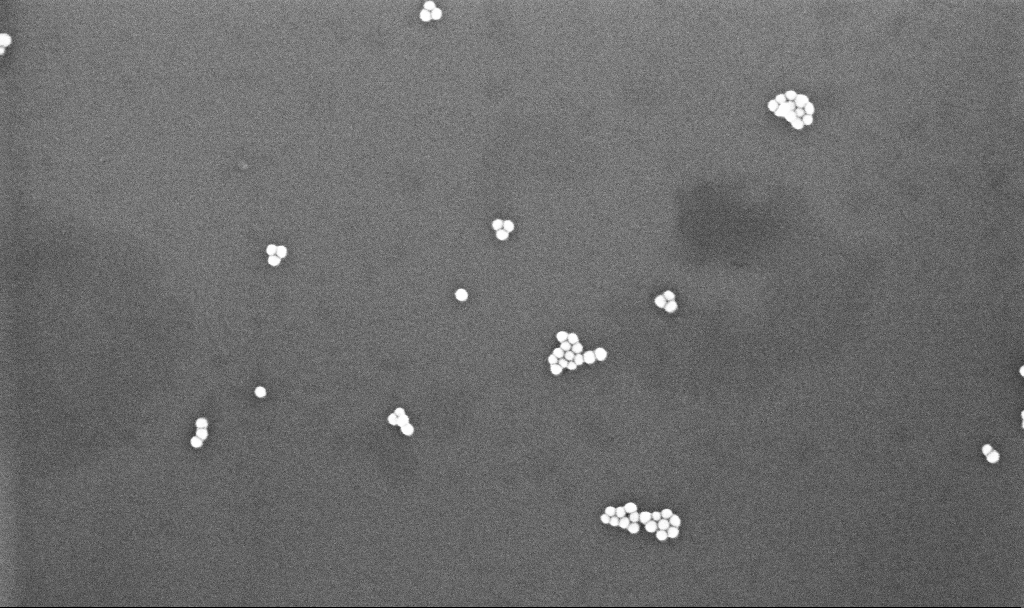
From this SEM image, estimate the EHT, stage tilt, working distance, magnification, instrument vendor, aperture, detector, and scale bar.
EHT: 10 kV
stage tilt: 0°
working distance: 3.3 mm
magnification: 174.82 K X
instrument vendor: Zeiss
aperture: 30 µm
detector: InLens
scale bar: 200 nm